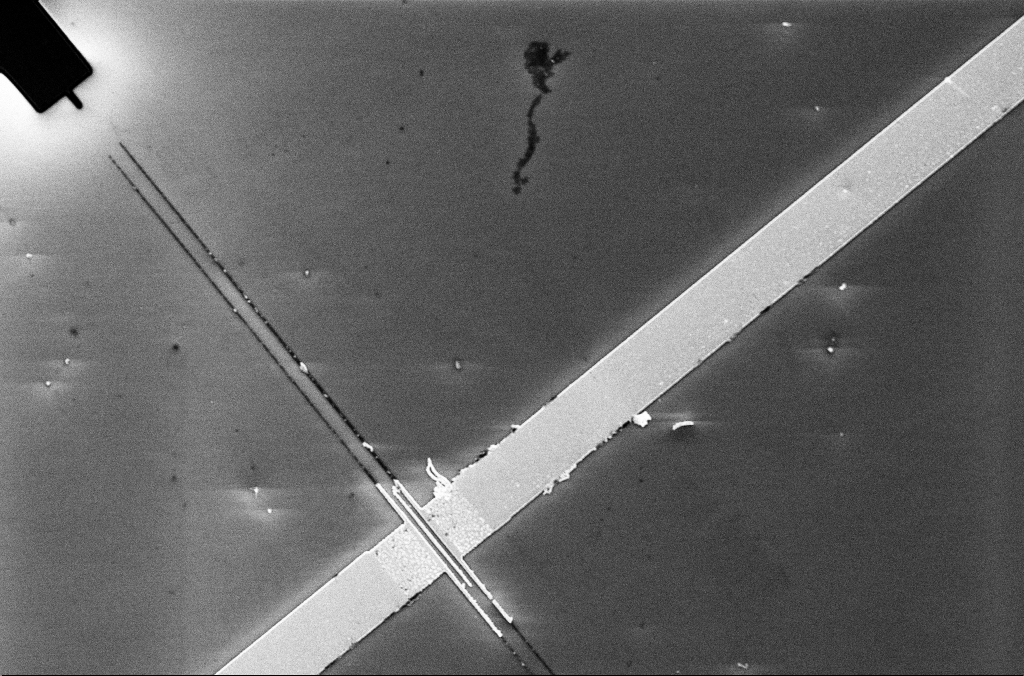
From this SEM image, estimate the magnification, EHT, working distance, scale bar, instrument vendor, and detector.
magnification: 4.74 K X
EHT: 5 kV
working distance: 3.3 mm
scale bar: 10000 nm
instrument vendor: Zeiss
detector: InLens